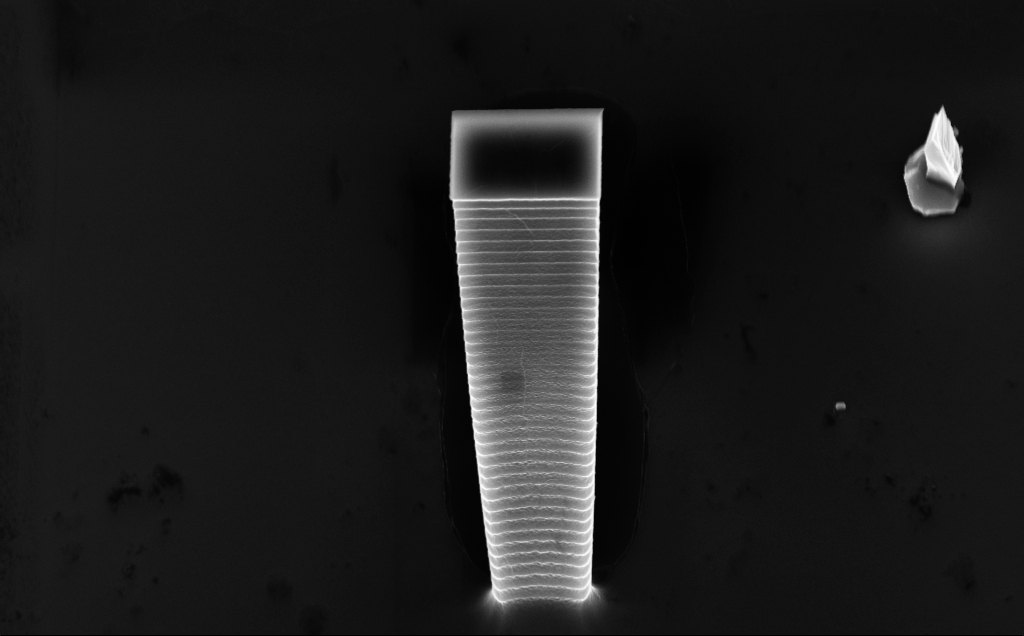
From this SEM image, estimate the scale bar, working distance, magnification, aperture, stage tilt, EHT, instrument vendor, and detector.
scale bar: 2000 nm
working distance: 5 mm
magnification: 11.59 K X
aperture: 30 µm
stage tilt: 45°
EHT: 10 kV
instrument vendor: Zeiss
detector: InLens